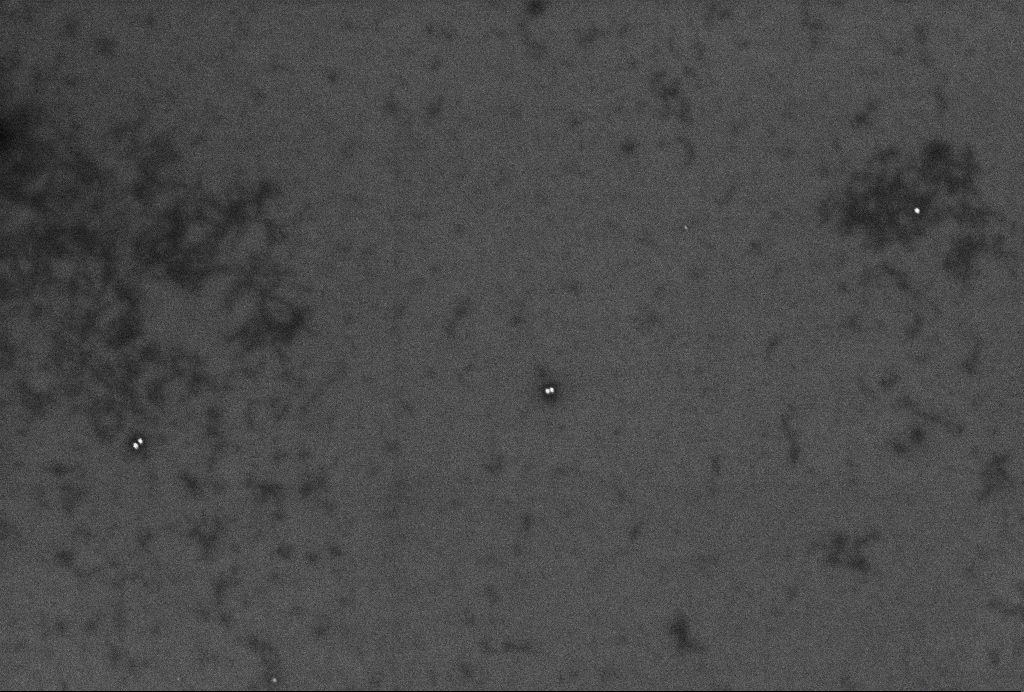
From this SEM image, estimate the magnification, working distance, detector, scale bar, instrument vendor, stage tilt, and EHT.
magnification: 62.65 K X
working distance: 3.3 mm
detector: InLens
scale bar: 200 nm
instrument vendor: Zeiss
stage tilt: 0°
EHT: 2 kV